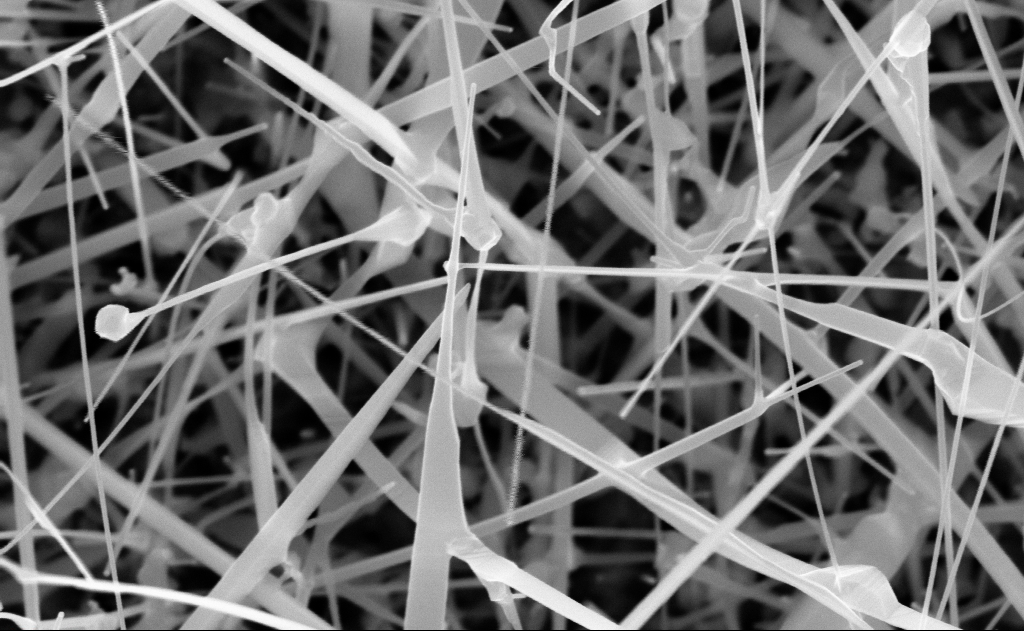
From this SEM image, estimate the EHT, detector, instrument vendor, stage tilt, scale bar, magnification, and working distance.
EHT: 10 kV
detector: InLens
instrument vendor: Zeiss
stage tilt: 0°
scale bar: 200 nm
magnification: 80 K X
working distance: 10 mm